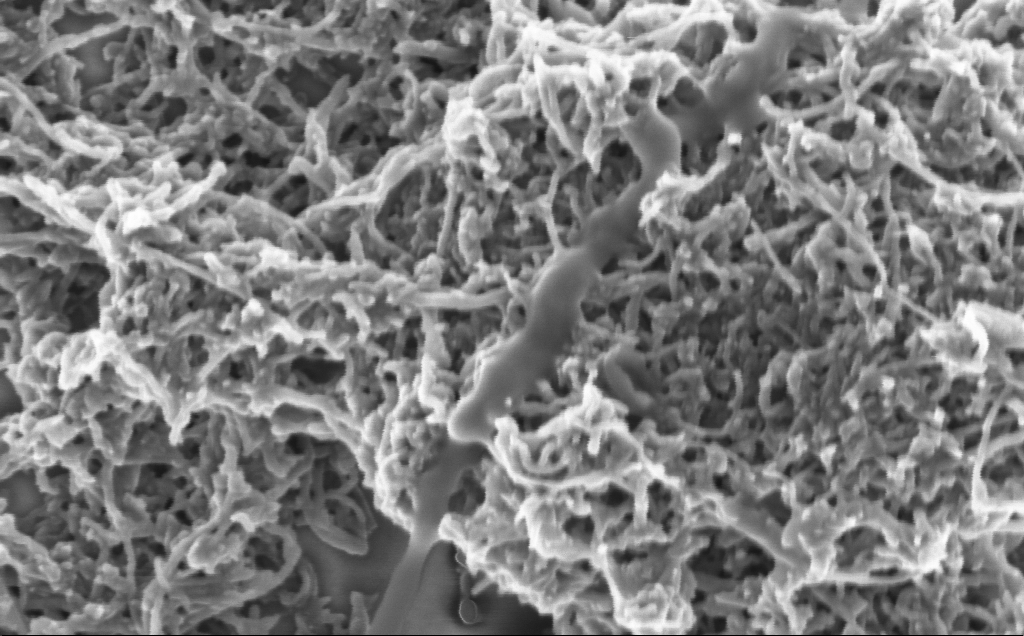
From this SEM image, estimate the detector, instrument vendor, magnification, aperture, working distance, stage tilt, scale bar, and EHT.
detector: InLens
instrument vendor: Zeiss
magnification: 100 K X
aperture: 30 µm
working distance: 7.1 mm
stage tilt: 0°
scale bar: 200 nm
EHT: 2 kV